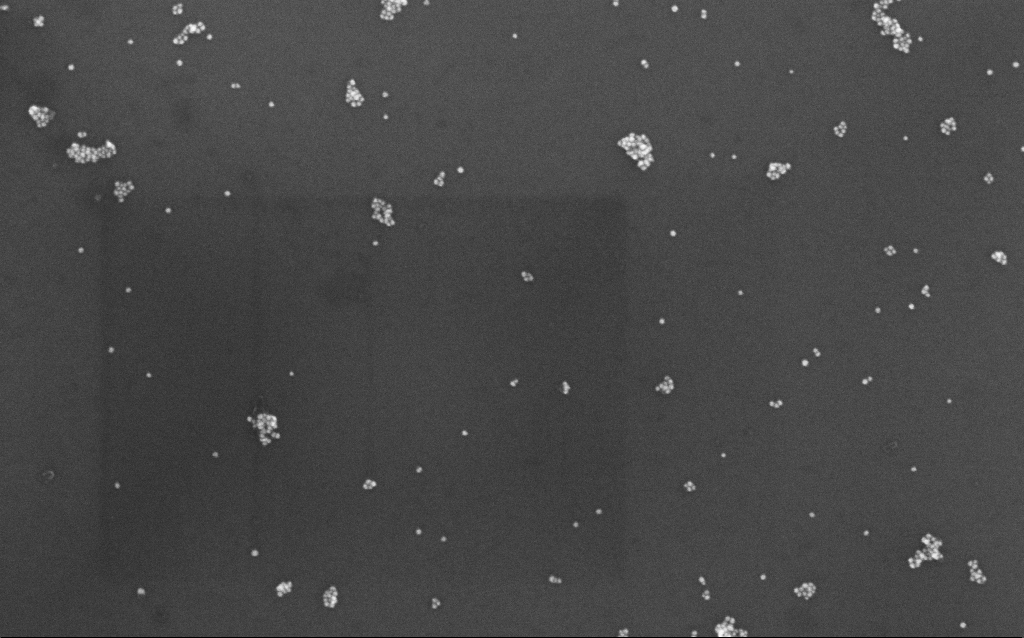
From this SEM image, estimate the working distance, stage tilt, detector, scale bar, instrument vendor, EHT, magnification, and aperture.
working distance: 7 mm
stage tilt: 0°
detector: InLens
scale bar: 200 nm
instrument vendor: Zeiss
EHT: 10 kV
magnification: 100 K X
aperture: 30 µm